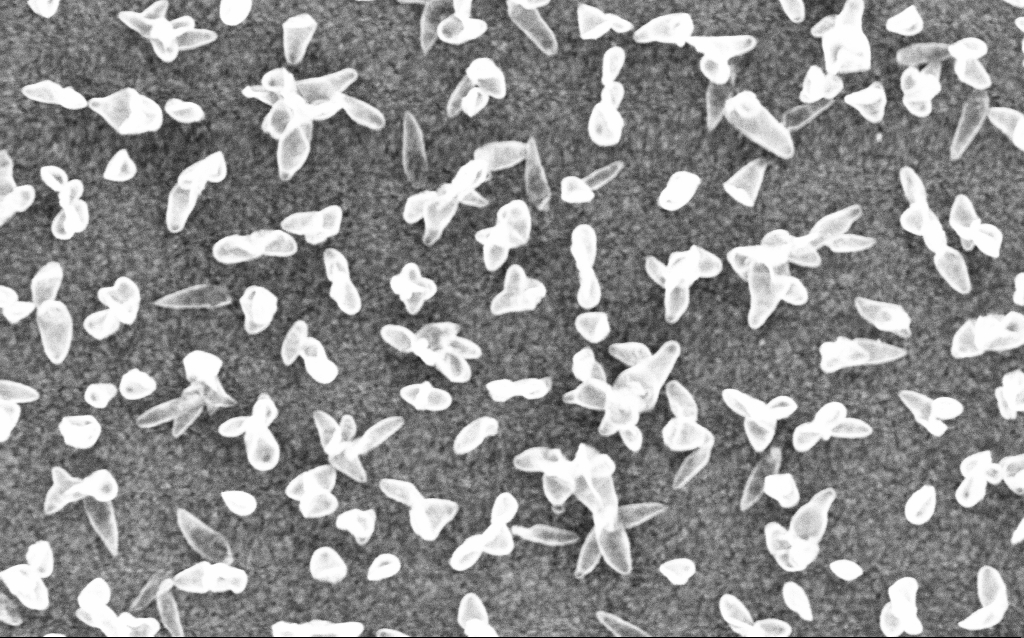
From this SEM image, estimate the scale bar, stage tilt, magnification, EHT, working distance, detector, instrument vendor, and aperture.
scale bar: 200 nm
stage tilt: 0°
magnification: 77.69 K X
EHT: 6 kV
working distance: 6 mm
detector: InLens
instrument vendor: Zeiss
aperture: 30 µm